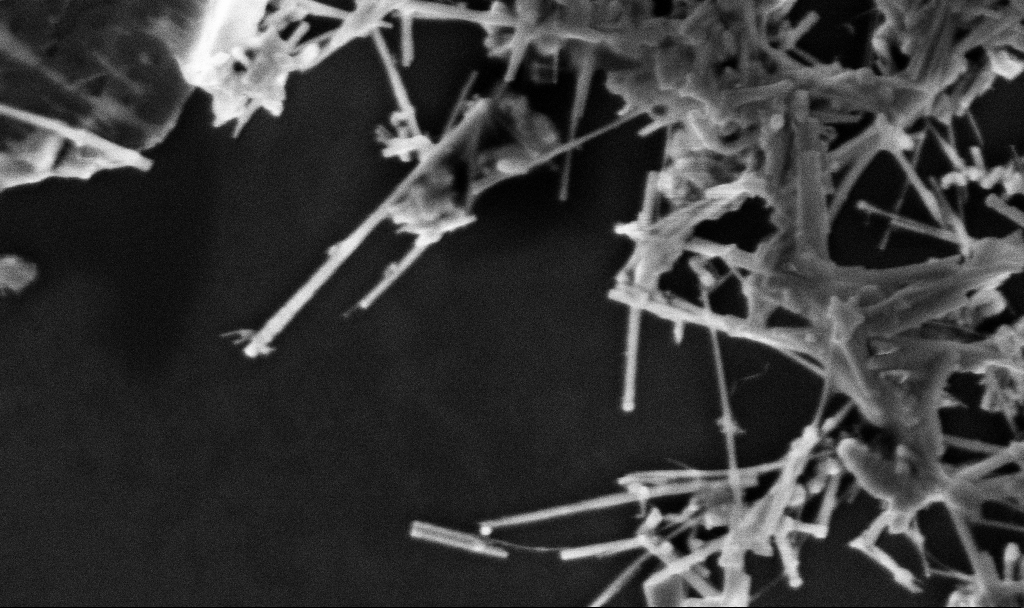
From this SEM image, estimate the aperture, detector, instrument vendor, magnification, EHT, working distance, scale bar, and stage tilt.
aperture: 30 µm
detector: InLens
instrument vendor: Zeiss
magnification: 79.74 K X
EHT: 3 kV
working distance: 3.3 mm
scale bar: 200 nm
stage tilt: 0°